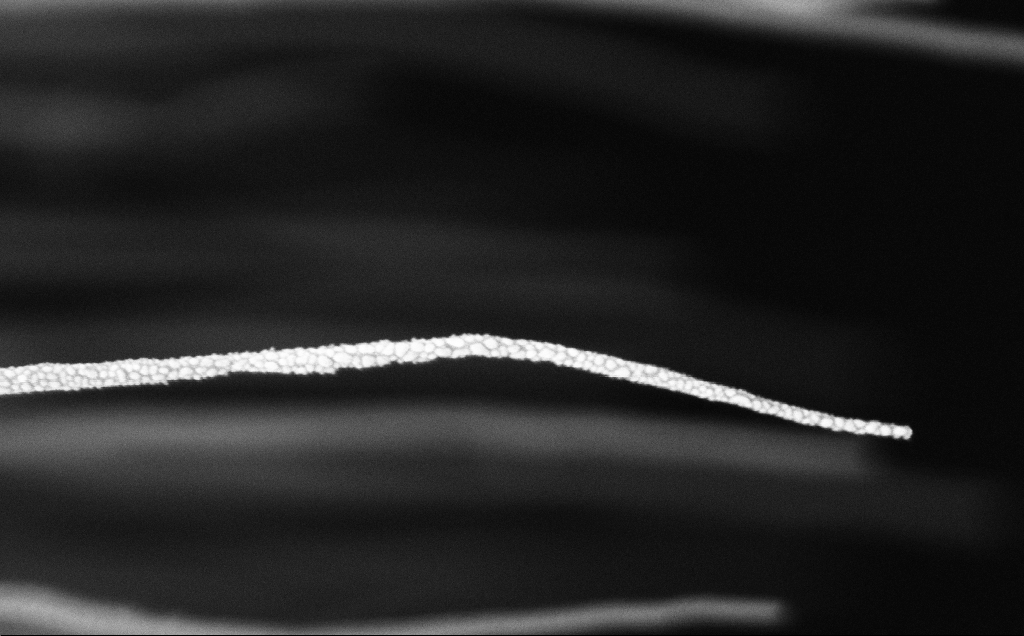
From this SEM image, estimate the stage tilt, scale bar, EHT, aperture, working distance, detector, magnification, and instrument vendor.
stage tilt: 0°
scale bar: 200 nm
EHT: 5 kV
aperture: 30 µm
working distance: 12 mm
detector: InLens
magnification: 89.56 K X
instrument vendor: Zeiss